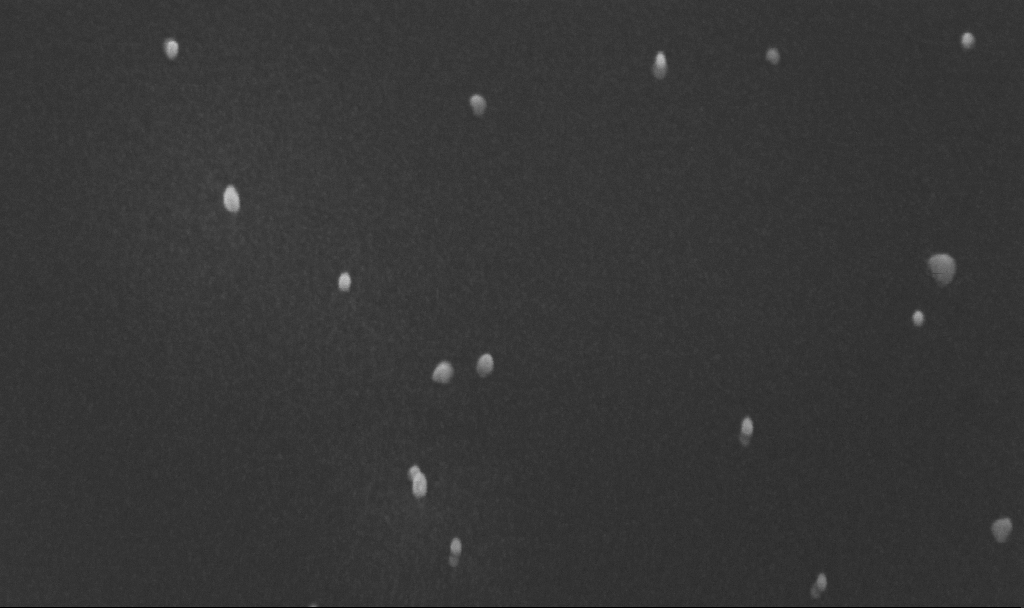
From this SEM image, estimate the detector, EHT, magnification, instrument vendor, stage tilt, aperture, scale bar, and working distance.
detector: InLens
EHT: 10 kV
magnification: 200 K X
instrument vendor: Zeiss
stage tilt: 45°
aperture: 30 µm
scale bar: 100 nm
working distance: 4.8 mm